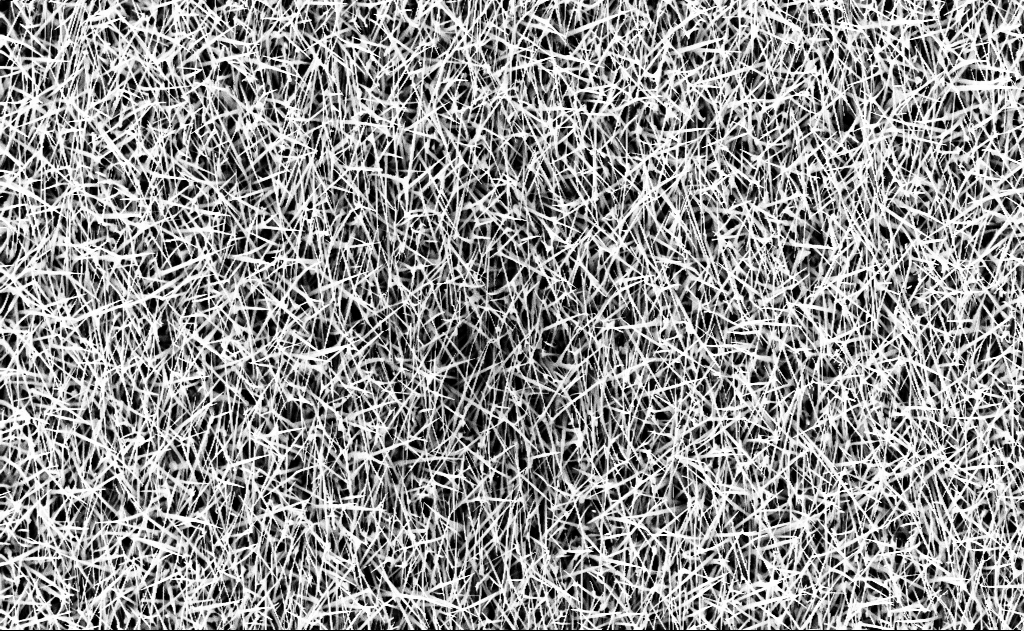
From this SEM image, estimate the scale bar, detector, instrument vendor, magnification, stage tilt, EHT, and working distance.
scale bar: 2000 nm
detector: InLens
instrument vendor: Zeiss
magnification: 10 K X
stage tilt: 0°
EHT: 10 kV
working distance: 16 mm